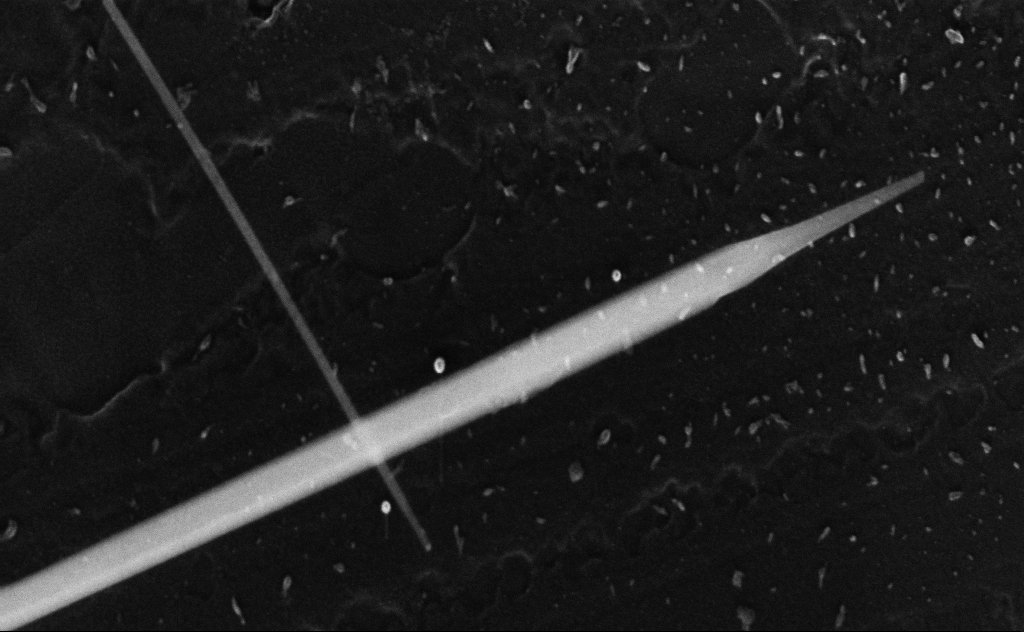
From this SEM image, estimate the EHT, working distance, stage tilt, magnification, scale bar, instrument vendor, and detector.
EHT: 20 kV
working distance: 8 mm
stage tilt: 0°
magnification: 118.89 K X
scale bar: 200 nm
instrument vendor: Zeiss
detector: InLens